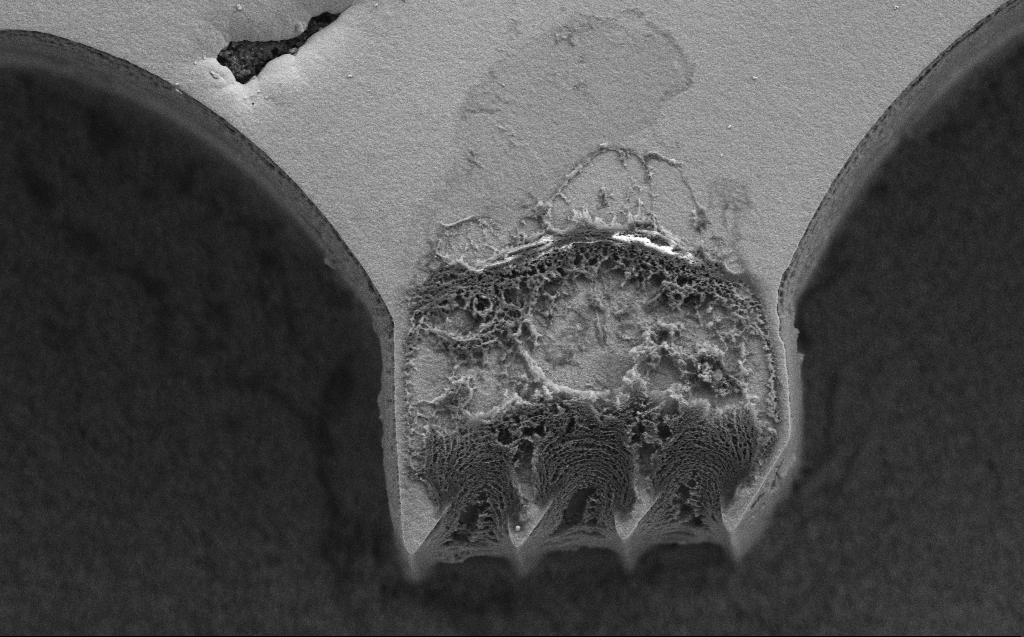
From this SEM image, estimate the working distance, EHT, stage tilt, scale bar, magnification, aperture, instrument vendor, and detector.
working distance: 7 mm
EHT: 5 kV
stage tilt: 0°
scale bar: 20000 nm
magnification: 3.46 K X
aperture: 30 µm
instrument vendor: Zeiss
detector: SE2